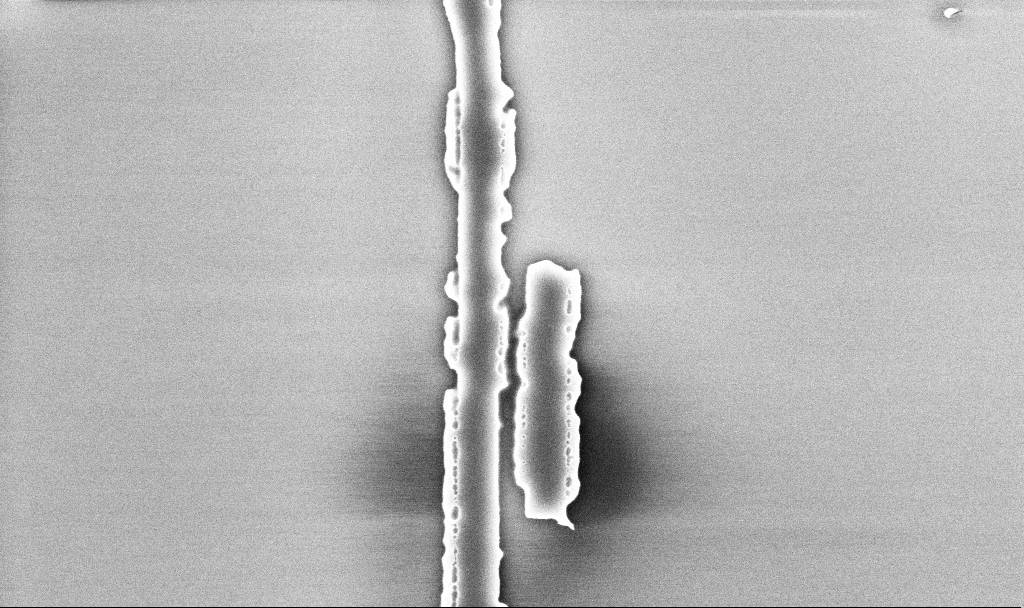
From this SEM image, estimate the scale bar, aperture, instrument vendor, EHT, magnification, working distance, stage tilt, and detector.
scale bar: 2000 nm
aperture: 30 µm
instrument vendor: Zeiss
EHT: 5 kV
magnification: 31 K X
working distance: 10.1 mm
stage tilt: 0°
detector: InLens